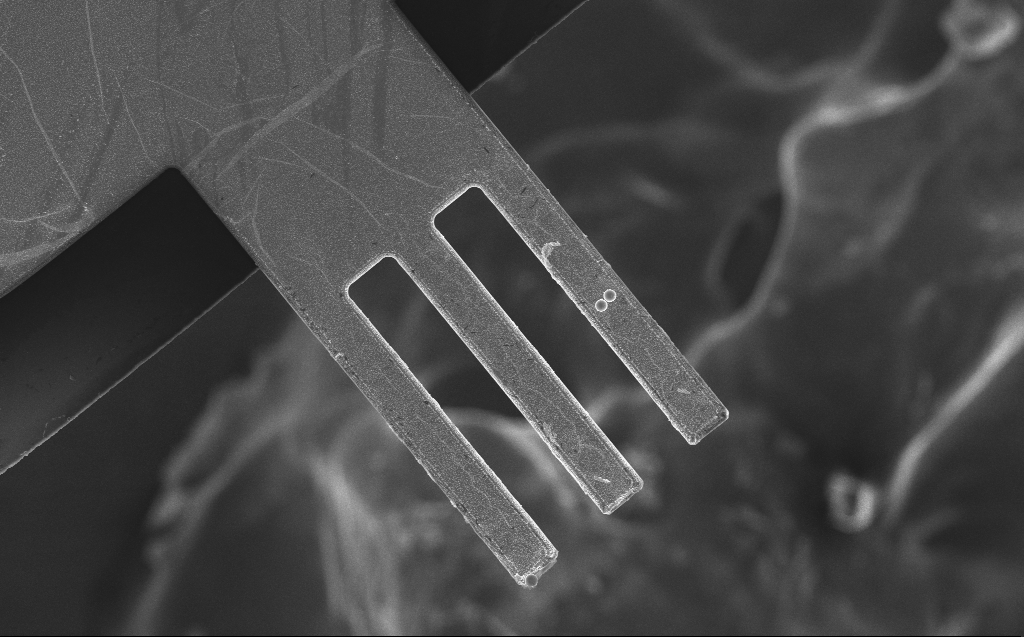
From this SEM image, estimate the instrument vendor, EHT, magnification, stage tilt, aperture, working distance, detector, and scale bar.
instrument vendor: Zeiss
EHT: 10 kV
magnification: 1.02 K X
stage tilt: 0°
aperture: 30 µm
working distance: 5 mm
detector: InLens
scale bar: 20000 nm